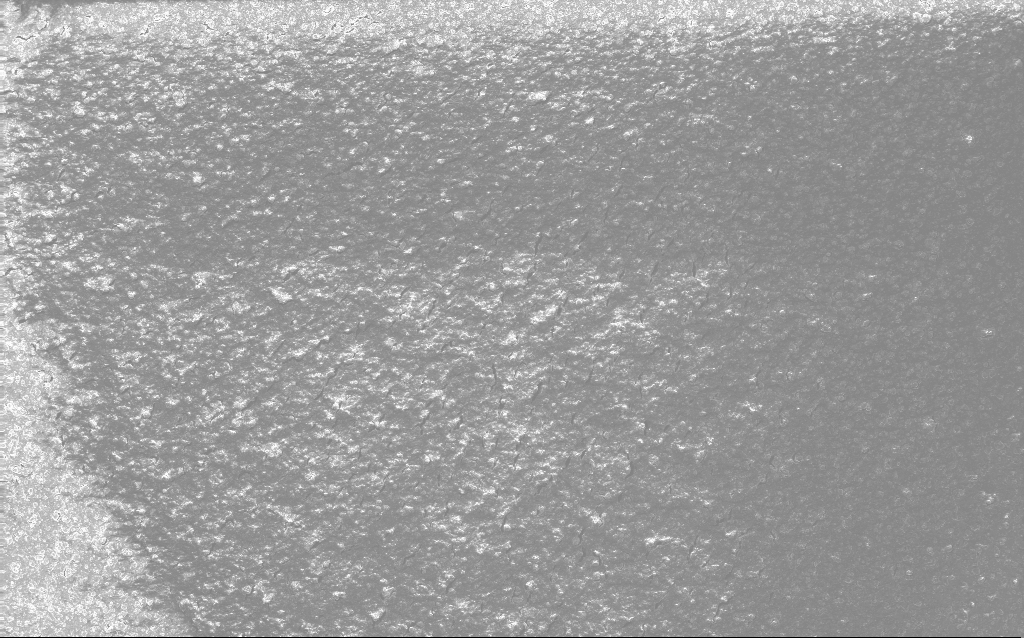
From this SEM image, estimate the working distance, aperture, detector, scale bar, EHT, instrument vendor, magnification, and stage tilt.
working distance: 2.6 mm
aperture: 30 µm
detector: InLens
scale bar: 100000 nm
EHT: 10 kV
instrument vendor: Zeiss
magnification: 0.235 K X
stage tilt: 0°